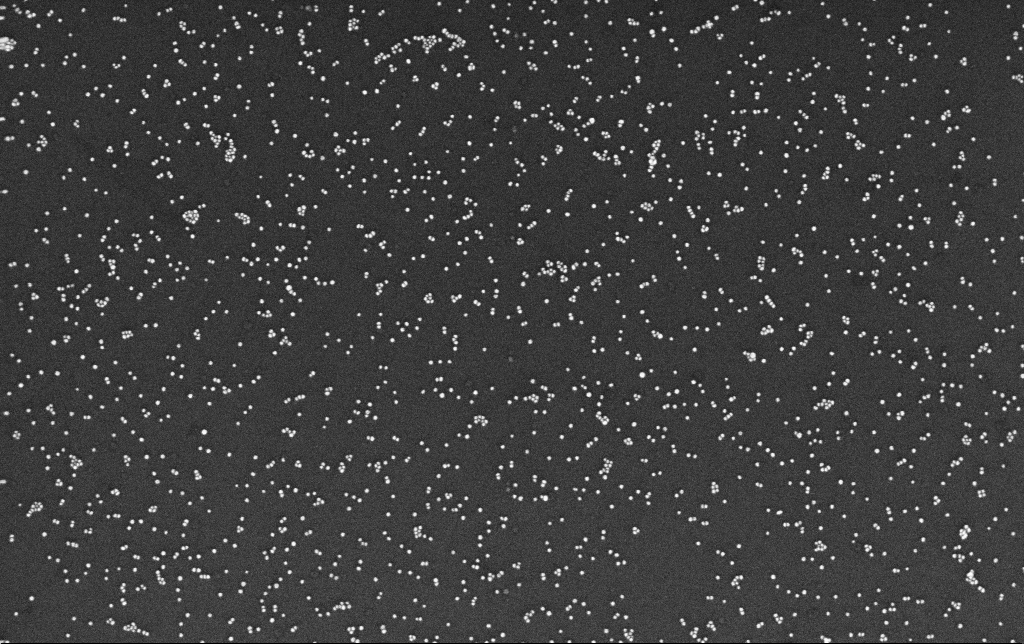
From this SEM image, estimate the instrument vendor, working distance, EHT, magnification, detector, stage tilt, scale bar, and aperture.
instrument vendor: Zeiss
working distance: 3.4 mm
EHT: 10 kV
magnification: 100 K X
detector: InLens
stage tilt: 0°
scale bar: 200 nm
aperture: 30 µm